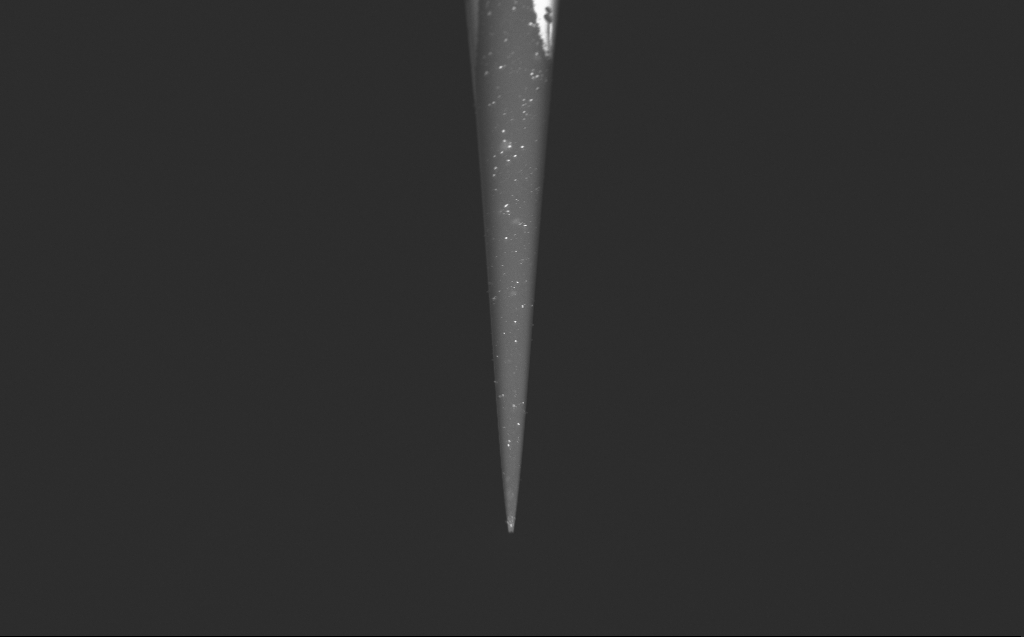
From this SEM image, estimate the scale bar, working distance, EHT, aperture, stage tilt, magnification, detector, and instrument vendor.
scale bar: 10000 nm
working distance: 4 mm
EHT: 5 kV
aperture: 30 µm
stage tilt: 45°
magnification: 4.47 K X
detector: InLens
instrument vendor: Zeiss